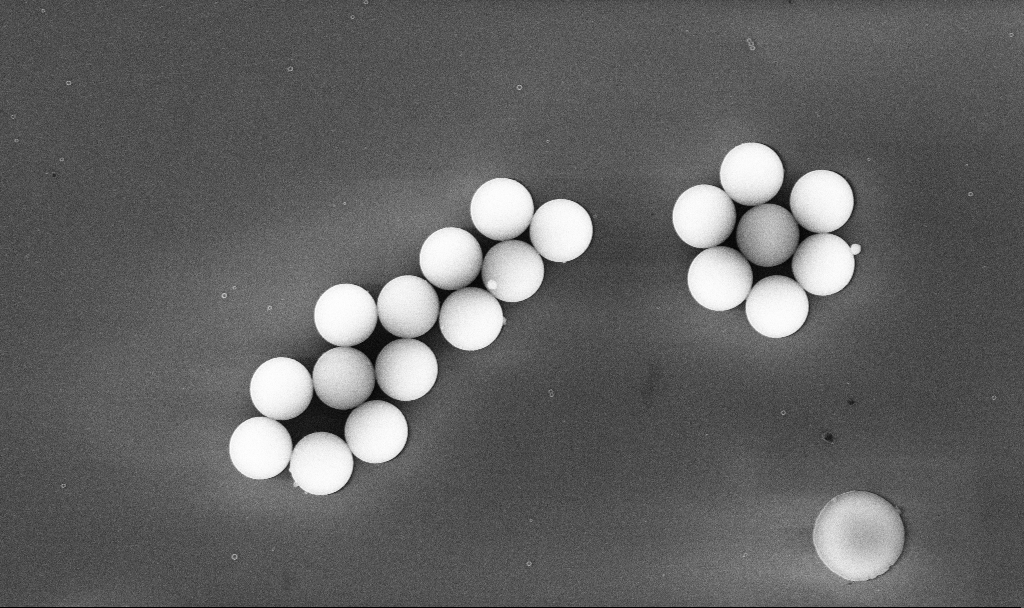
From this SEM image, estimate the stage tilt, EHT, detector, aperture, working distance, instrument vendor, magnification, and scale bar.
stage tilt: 0°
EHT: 10 kV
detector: InLens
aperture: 30 µm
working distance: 5.2 mm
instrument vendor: Zeiss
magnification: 11.2 K X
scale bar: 2000 nm